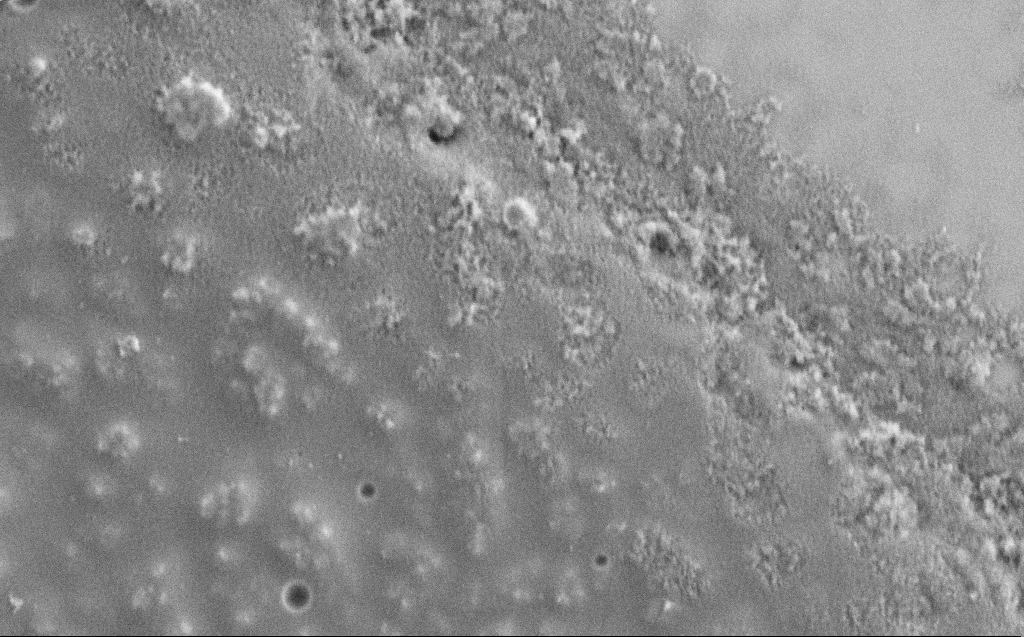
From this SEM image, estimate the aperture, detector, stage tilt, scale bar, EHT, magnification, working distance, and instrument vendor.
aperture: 30 µm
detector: SE2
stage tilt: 0°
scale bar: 1000 nm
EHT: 1.2 kV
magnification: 61.65 K X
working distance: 4 mm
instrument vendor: Zeiss